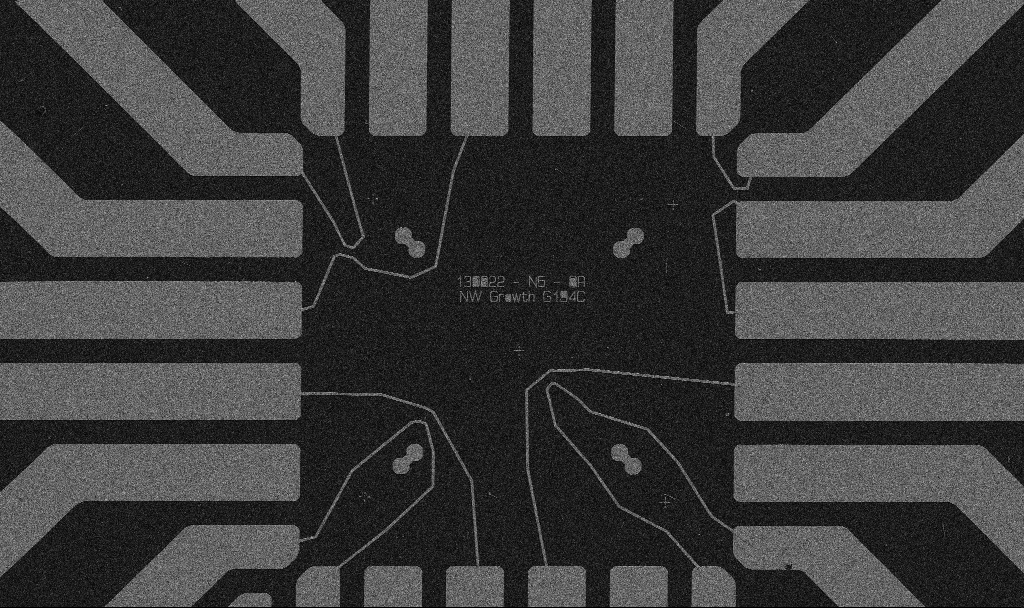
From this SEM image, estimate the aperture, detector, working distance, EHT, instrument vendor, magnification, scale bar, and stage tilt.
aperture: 30 µm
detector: SE2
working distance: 10.7 mm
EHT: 5 kV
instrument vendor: Zeiss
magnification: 1 K X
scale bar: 20000 nm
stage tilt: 0°